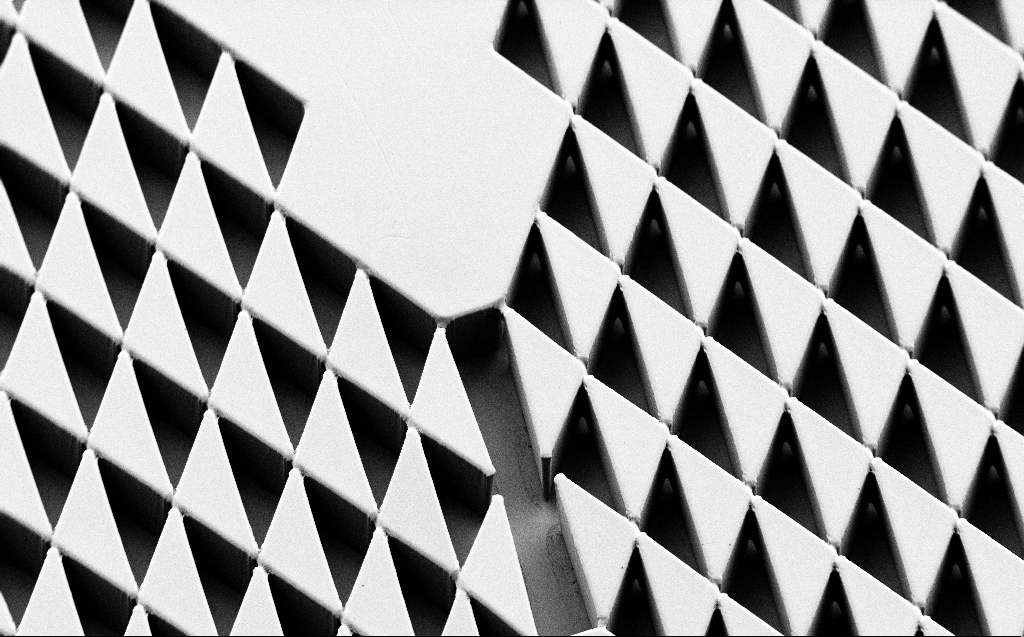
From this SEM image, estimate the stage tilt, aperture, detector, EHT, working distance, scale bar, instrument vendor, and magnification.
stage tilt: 45°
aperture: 30 µm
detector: SE2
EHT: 1 kV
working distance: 6 mm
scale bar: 100000 nm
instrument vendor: Zeiss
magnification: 0.639 K X